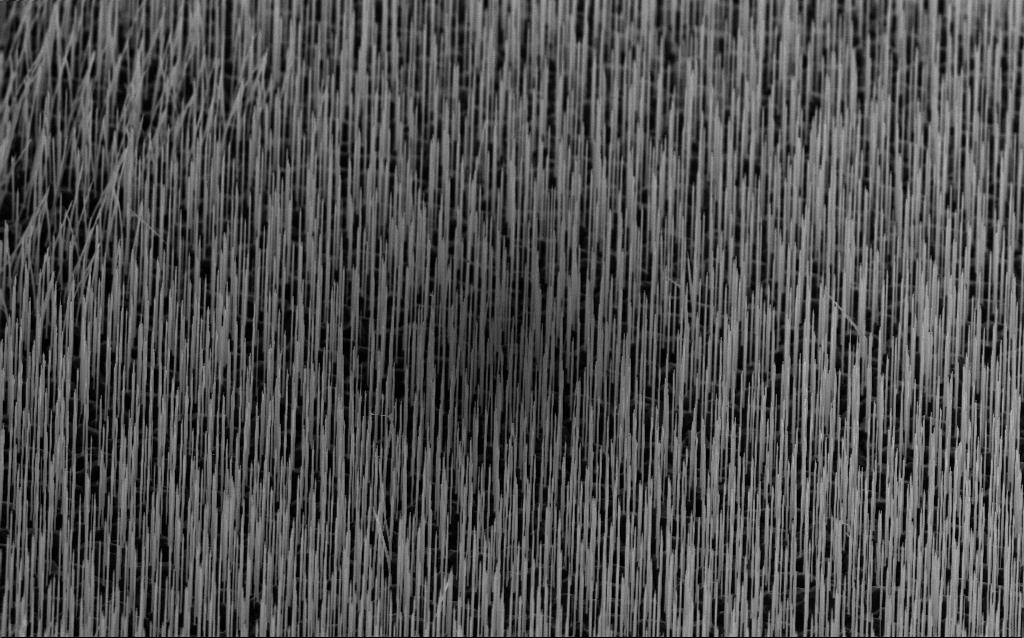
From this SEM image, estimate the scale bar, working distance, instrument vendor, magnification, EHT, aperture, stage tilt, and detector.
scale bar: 2000 nm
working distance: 6.3 mm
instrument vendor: Zeiss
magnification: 10 K X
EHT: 5 kV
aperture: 30 µm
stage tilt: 40°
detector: InLens